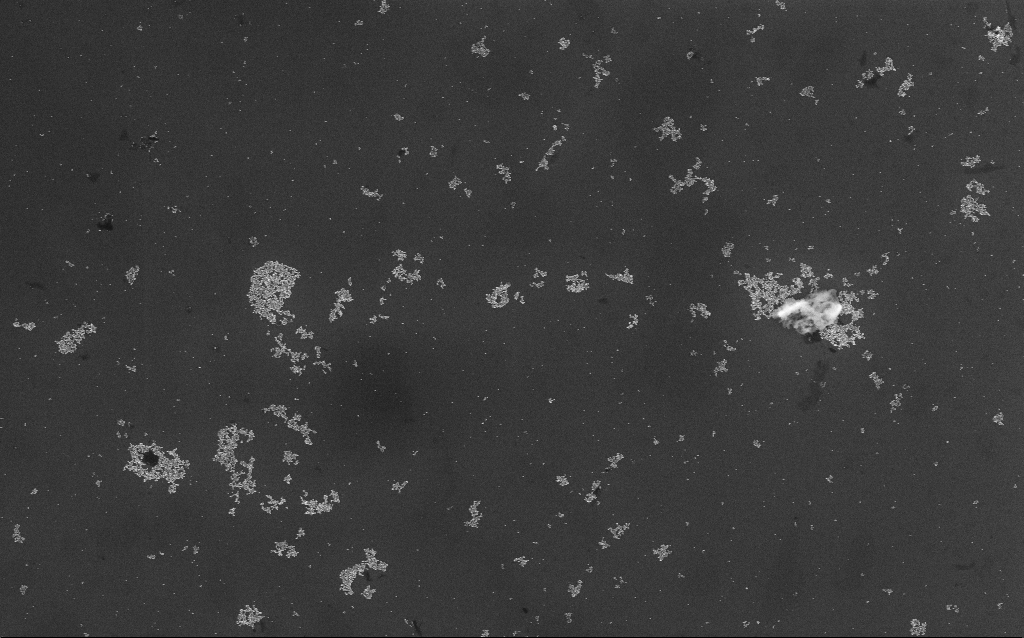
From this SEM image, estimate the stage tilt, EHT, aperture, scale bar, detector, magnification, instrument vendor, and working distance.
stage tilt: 0°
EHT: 10 kV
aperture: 30 µm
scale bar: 1000 nm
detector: InLens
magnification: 18.45 K X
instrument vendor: Zeiss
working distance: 7 mm